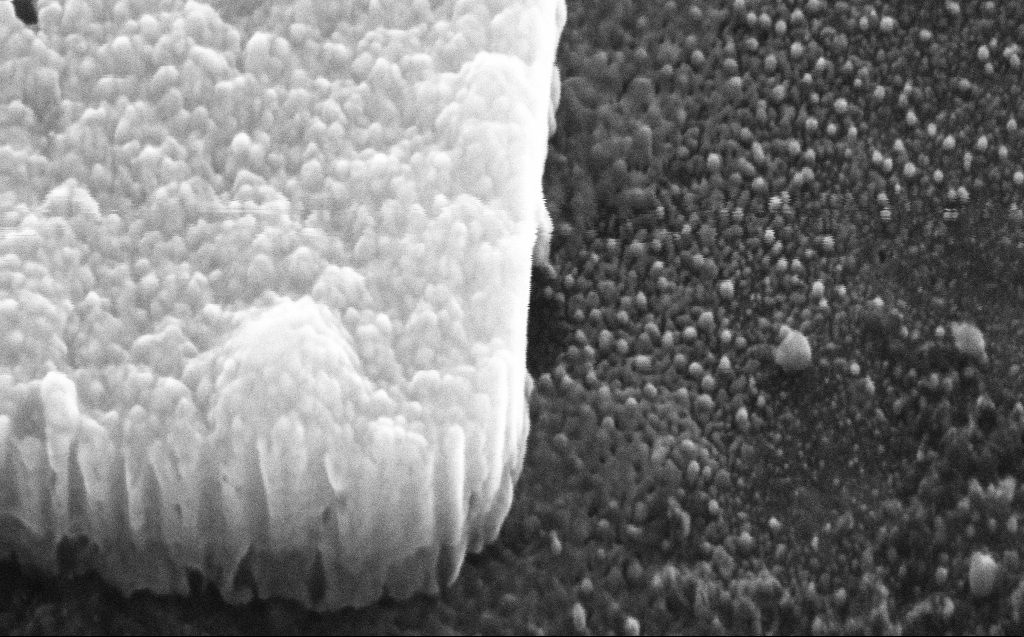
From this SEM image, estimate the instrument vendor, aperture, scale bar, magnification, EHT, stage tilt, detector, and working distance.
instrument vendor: Zeiss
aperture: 30 µm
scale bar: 200 nm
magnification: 342.18 K X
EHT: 30 kV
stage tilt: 45°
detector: SE2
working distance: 5 mm